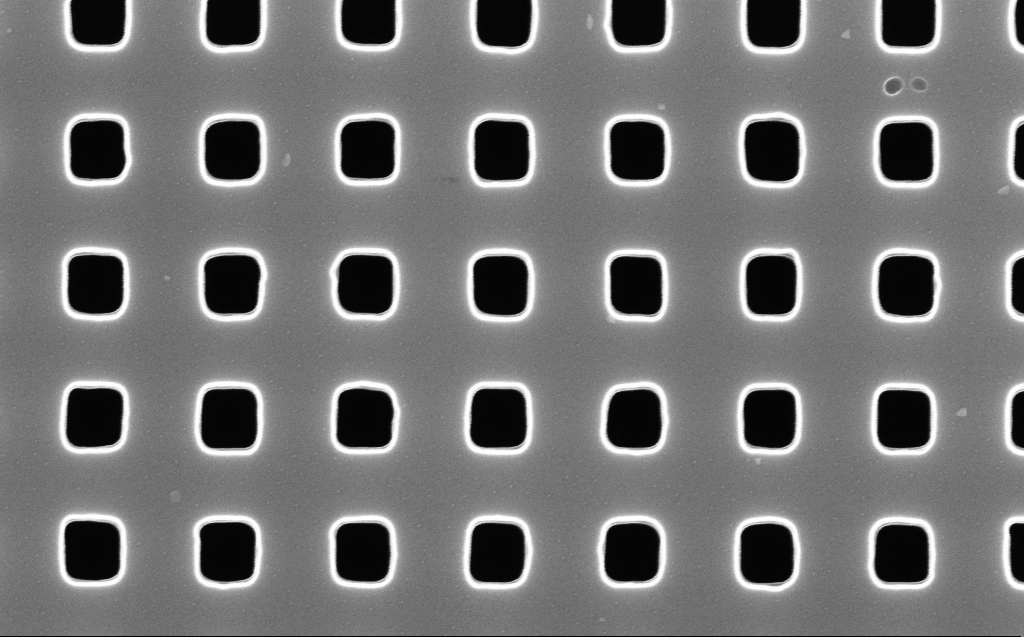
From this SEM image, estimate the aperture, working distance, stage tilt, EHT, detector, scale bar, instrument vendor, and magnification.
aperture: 30 µm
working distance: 6 mm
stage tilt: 0°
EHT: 10 kV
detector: InLens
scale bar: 200 nm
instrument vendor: Zeiss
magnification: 100 K X